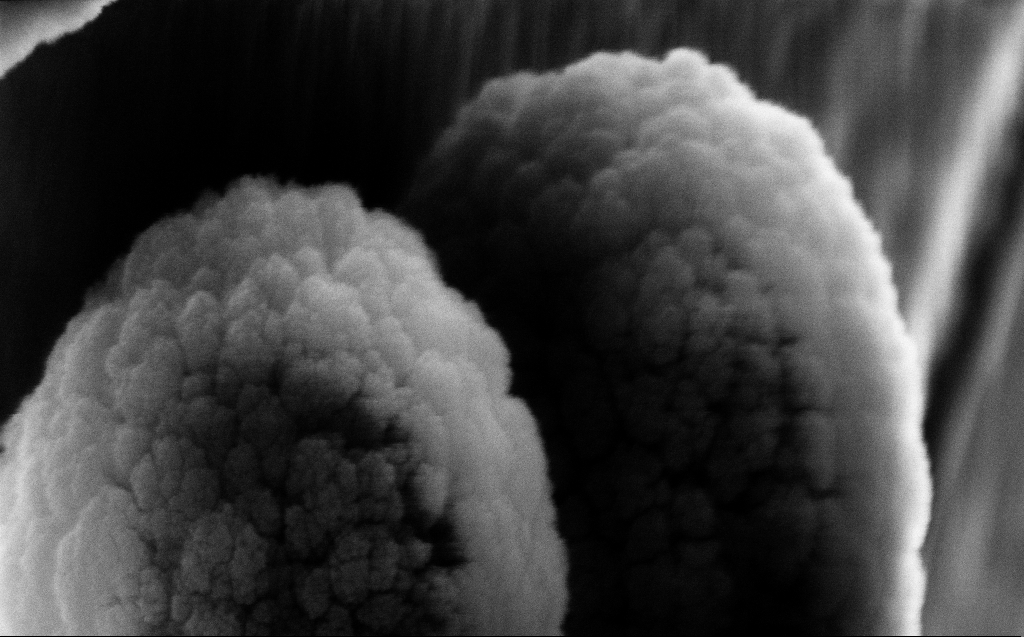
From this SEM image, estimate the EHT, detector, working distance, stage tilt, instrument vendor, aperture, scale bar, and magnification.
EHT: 10 kV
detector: InLens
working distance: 5 mm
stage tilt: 45°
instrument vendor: Zeiss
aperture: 30 µm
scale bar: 200 nm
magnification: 148.68 K X